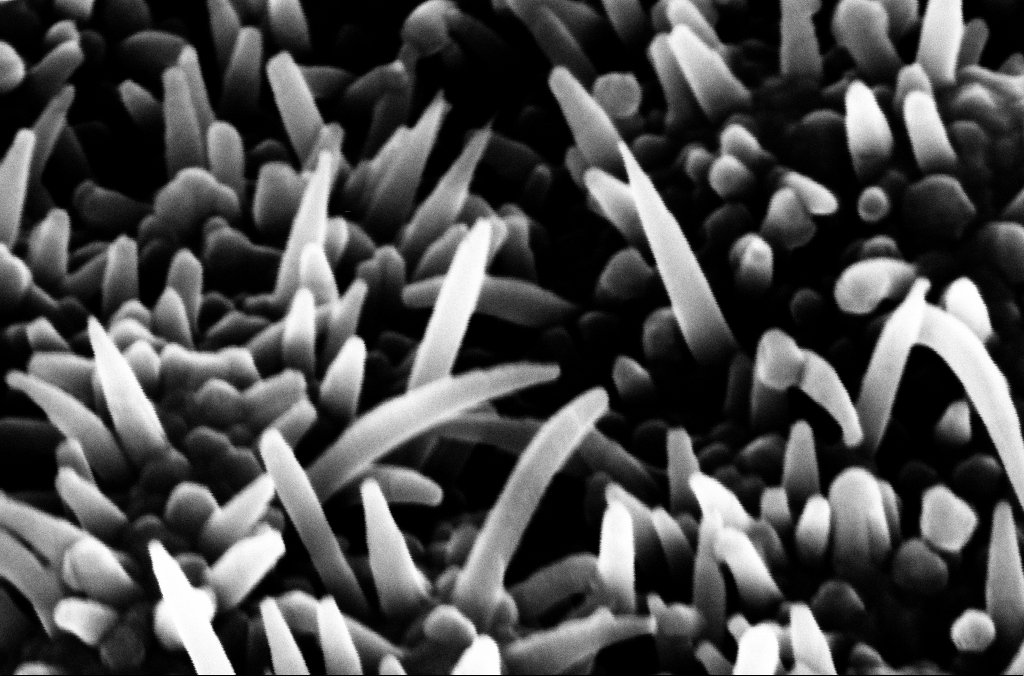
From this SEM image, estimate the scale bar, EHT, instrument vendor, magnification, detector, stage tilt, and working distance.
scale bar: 200 nm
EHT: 10 kV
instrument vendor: Zeiss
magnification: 241.53 K X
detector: InLens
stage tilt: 45°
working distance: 6.6 mm